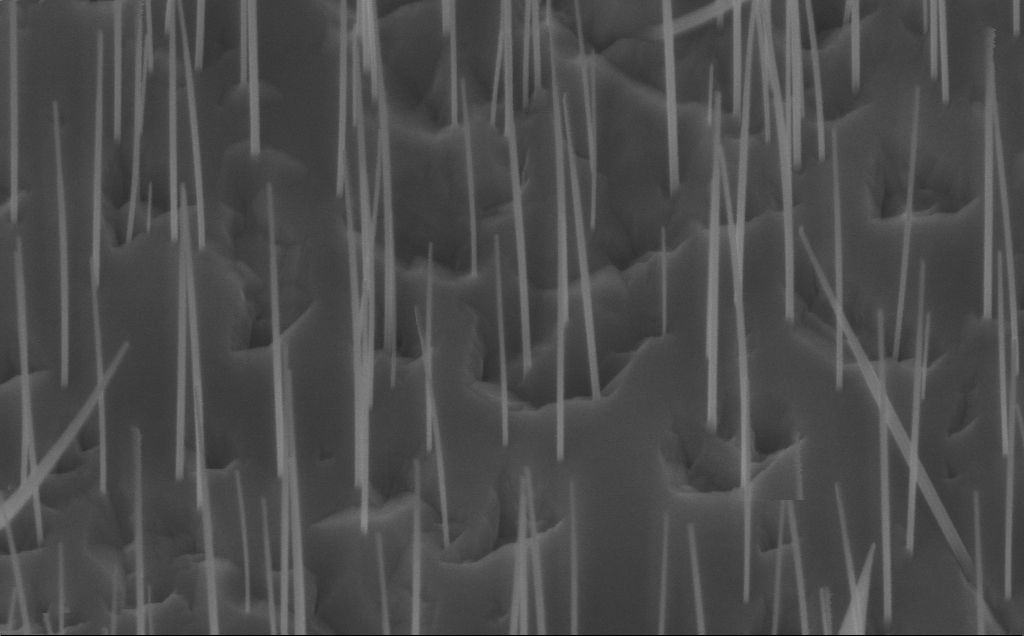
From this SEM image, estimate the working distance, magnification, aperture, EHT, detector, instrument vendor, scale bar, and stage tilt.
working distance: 7 mm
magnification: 80 K X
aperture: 30 µm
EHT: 10 kV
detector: InLens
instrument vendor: Zeiss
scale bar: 200 nm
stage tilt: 45°